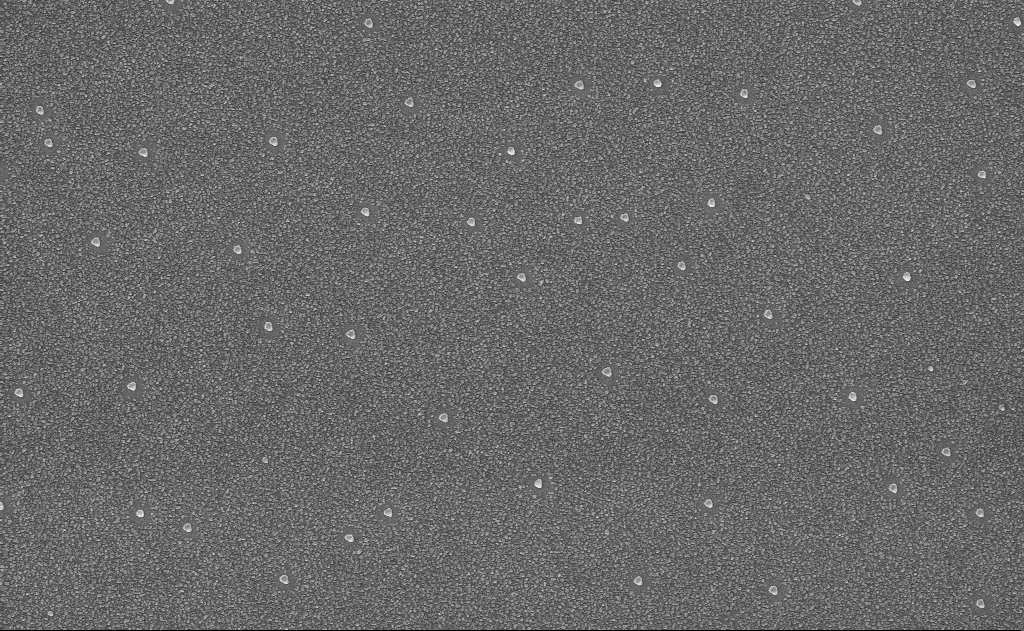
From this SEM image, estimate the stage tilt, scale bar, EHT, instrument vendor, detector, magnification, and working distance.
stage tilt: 0°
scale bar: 2000 nm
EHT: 10 kV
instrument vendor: Zeiss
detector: InLens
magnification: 20 K X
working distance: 15 mm